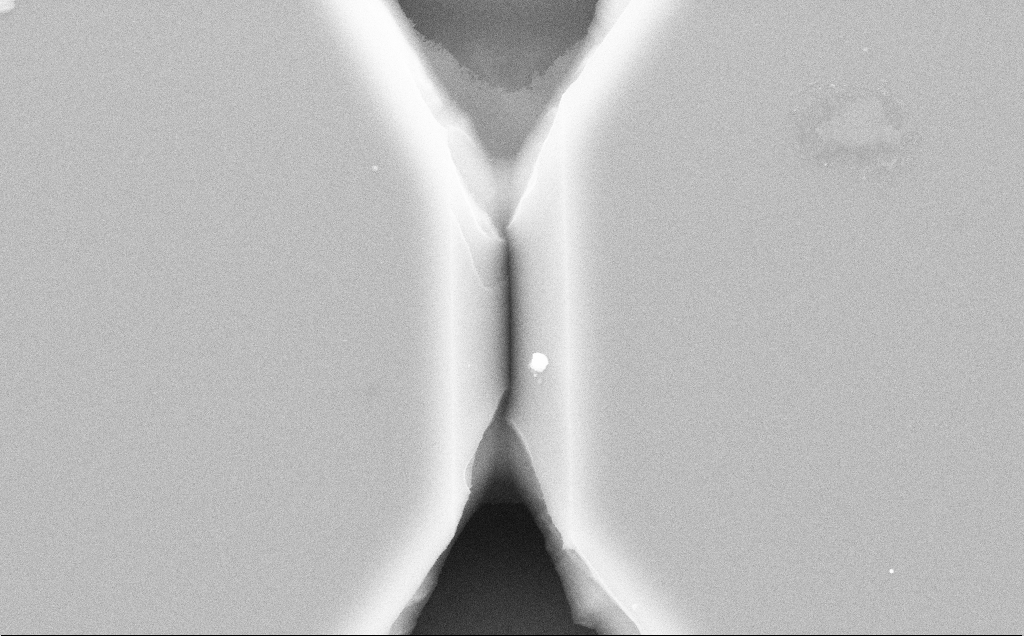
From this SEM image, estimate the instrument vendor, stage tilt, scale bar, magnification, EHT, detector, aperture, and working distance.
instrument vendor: Zeiss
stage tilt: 0°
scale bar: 1000 nm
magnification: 19.23 K X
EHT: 10 kV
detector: SE2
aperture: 30 µm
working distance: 10 mm